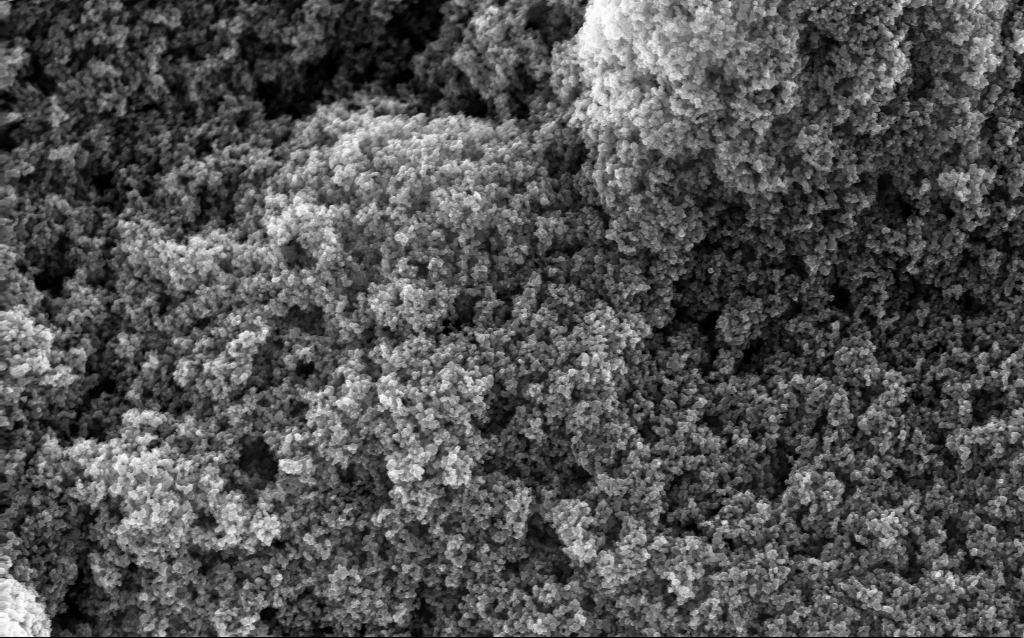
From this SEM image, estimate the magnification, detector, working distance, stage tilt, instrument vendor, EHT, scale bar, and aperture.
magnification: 65.04 K X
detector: InLens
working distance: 2.7 mm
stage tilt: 0°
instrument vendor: Zeiss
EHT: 10 kV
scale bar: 1000 nm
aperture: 30 µm